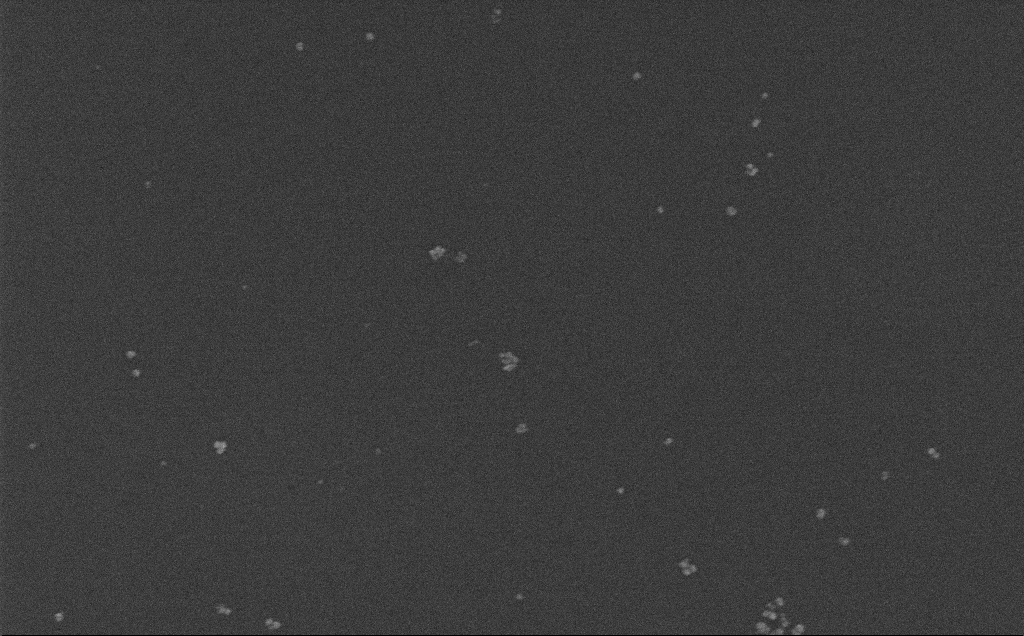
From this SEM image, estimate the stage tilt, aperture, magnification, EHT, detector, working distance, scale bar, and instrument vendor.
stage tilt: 0°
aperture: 30 µm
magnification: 100 K X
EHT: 10 kV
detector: InLens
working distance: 3.7 mm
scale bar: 200 nm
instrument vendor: Zeiss